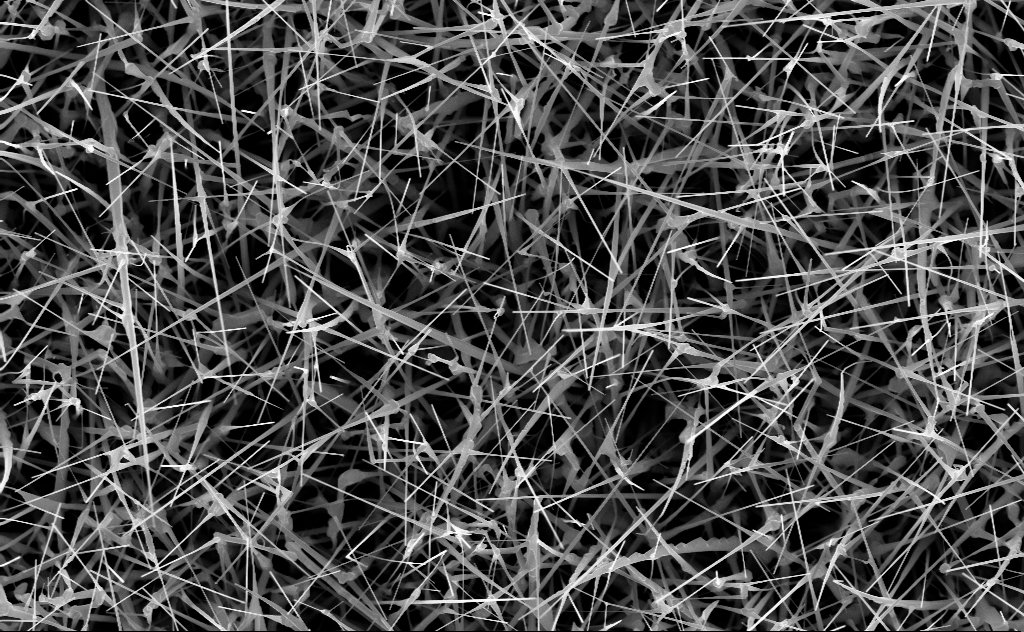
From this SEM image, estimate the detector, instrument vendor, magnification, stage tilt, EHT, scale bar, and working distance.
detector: InLens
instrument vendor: Zeiss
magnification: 20 K X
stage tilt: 0°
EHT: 10 kV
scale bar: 2000 nm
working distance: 6 mm